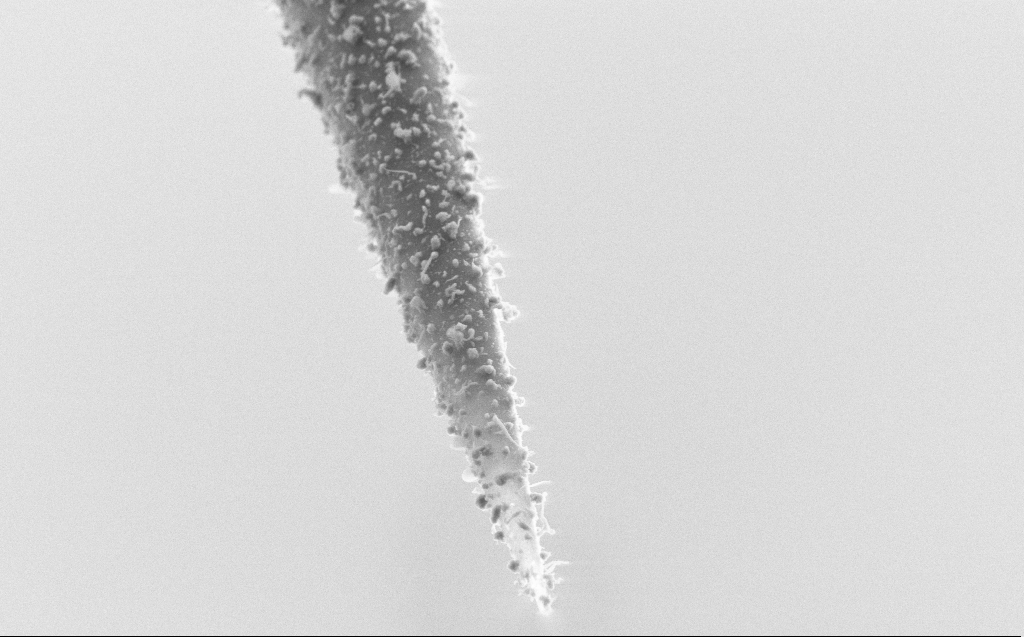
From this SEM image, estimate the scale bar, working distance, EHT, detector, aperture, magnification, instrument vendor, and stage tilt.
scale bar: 2000 nm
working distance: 4 mm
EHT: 5 kV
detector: SE2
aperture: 30 µm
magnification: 19.04 K X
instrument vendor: Zeiss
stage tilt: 45°